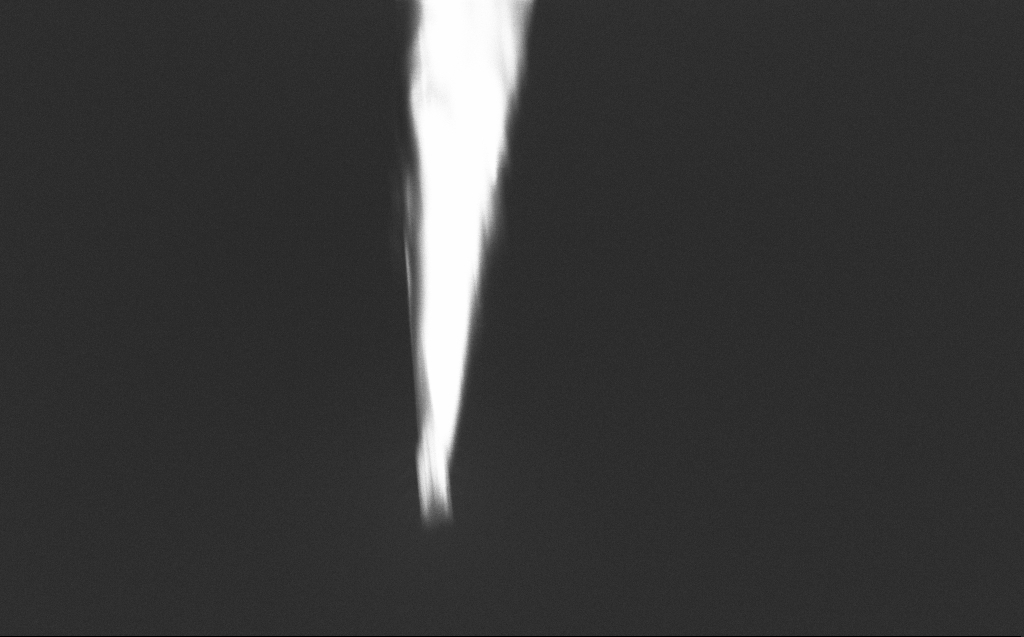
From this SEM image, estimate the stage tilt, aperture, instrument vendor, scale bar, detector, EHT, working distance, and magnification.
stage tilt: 45°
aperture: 20 µm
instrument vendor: Zeiss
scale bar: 2000 nm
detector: InLens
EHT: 2 kV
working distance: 4 mm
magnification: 22.85 K X